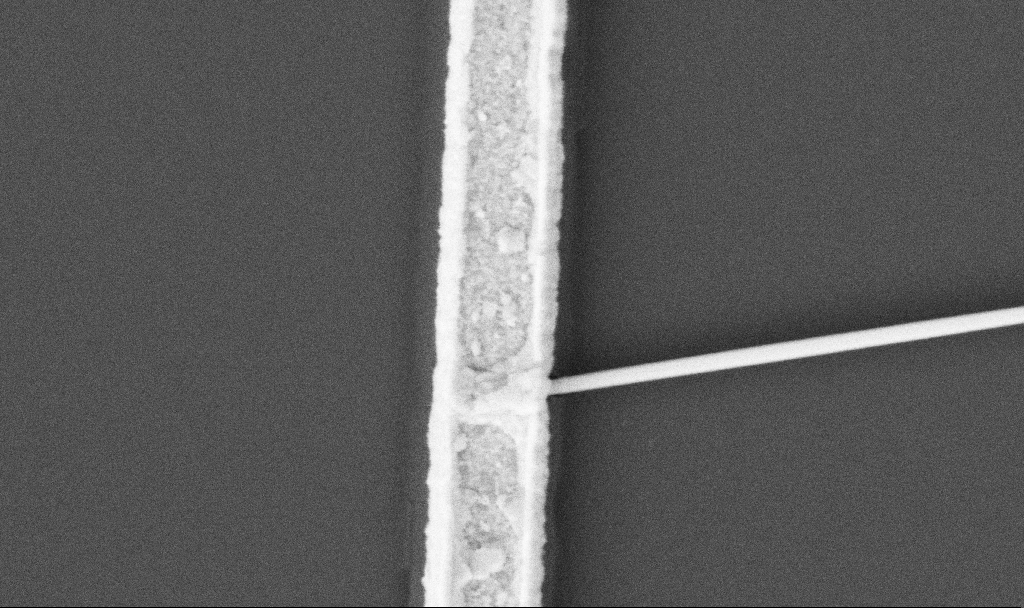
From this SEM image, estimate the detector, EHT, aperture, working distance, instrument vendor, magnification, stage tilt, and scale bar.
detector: SE2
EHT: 5 kV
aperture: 30 µm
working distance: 10.7 mm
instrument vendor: Zeiss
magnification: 60 K X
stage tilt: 0°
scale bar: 1000 nm